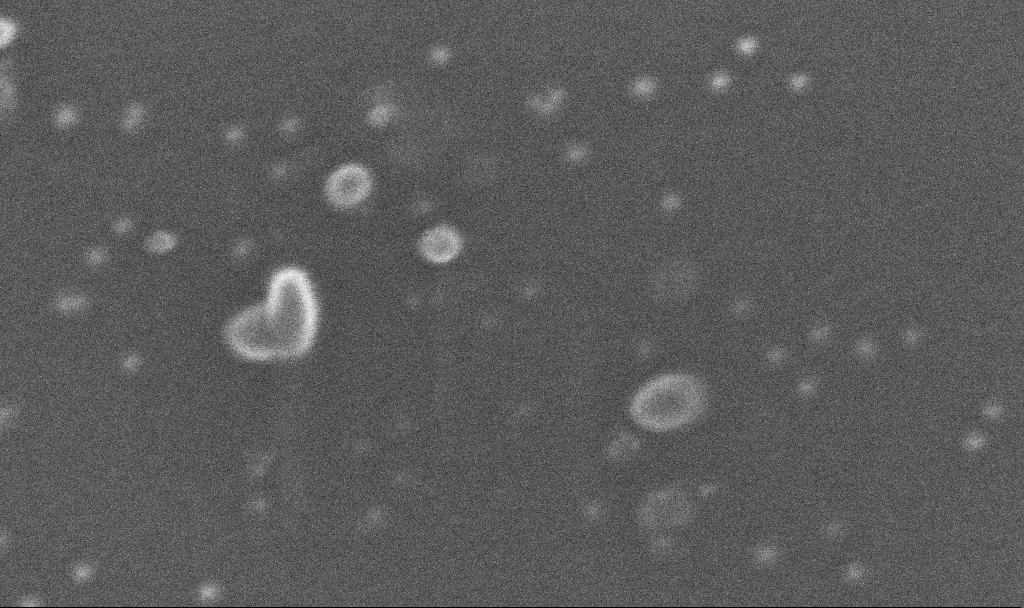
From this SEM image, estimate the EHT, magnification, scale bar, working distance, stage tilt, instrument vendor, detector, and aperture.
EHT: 10 kV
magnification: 537.09 K X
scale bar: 100 nm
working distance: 3.3 mm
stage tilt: -0°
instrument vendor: Zeiss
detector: InLens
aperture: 30 µm